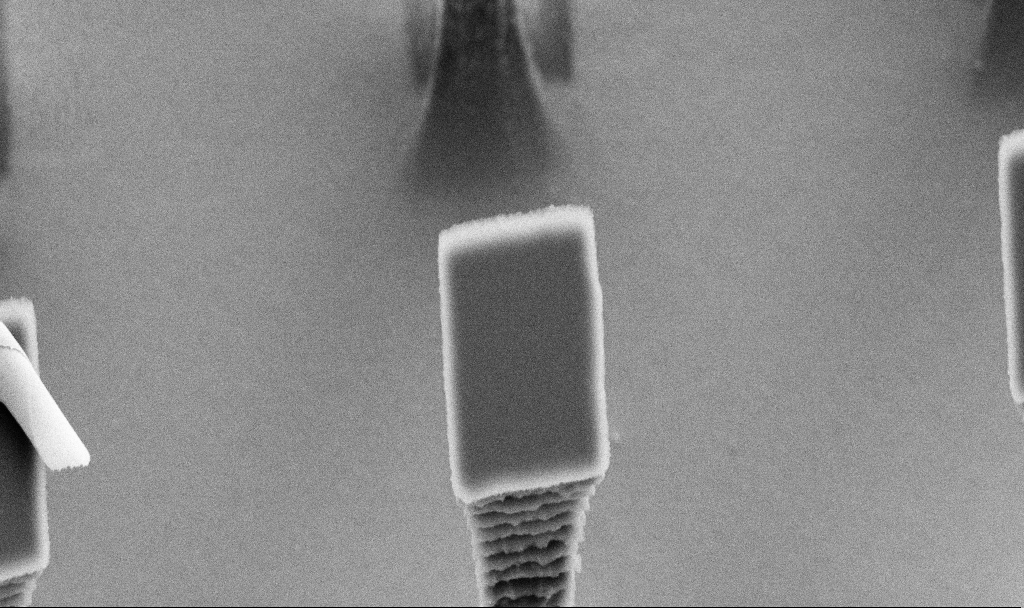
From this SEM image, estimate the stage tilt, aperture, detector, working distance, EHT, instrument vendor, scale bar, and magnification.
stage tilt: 30°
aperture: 30 µm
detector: SE2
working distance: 9.5 mm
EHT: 5 kV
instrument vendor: Zeiss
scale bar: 2000 nm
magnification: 17.14 K X